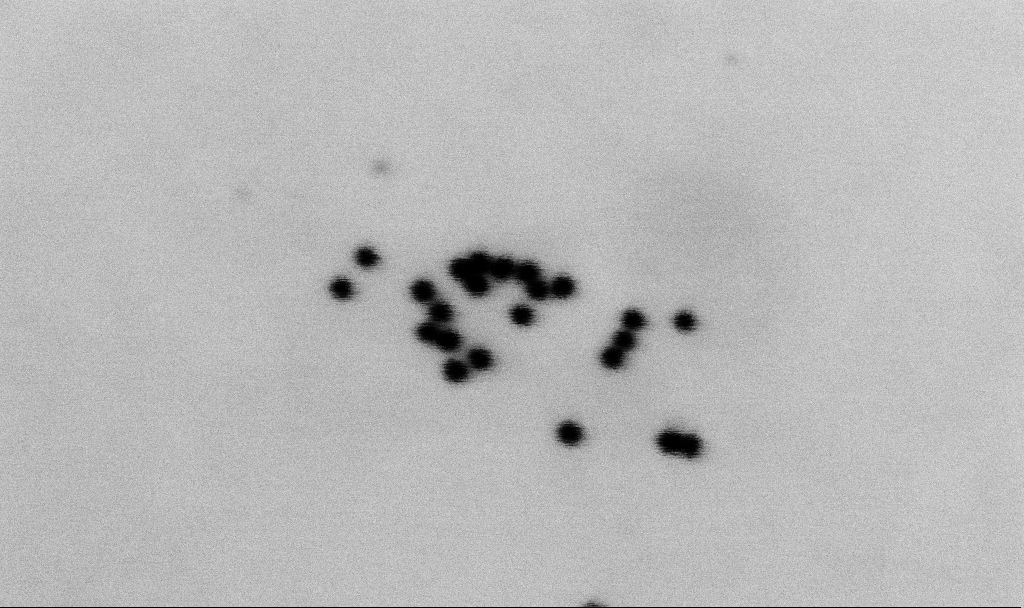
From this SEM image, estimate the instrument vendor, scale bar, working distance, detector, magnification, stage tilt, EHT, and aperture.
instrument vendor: Zeiss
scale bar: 100 nm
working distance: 5.5 mm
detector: SE2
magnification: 368.85 K X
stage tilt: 0°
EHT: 2 kV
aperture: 30 µm